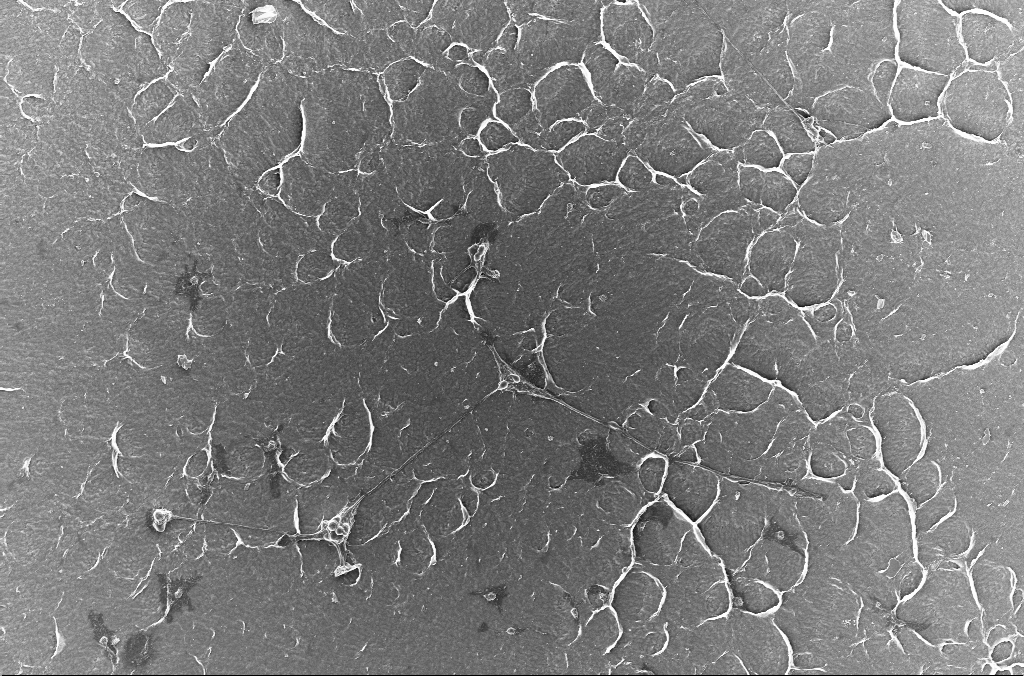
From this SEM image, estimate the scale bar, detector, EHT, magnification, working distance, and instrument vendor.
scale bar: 100000 nm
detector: InLens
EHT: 5 kV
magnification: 0.5 K X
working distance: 2.9 mm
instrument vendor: Zeiss